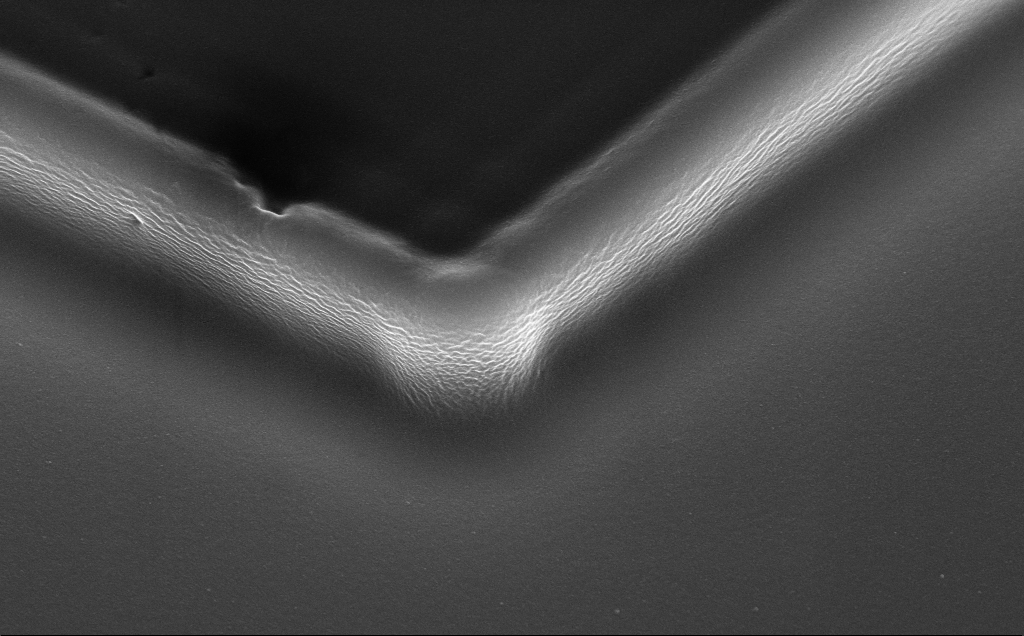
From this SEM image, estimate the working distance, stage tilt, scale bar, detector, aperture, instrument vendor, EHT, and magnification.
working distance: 6 mm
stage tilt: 35°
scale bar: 1000 nm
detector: InLens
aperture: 30 µm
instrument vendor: Zeiss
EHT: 7 kV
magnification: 46.7 K X